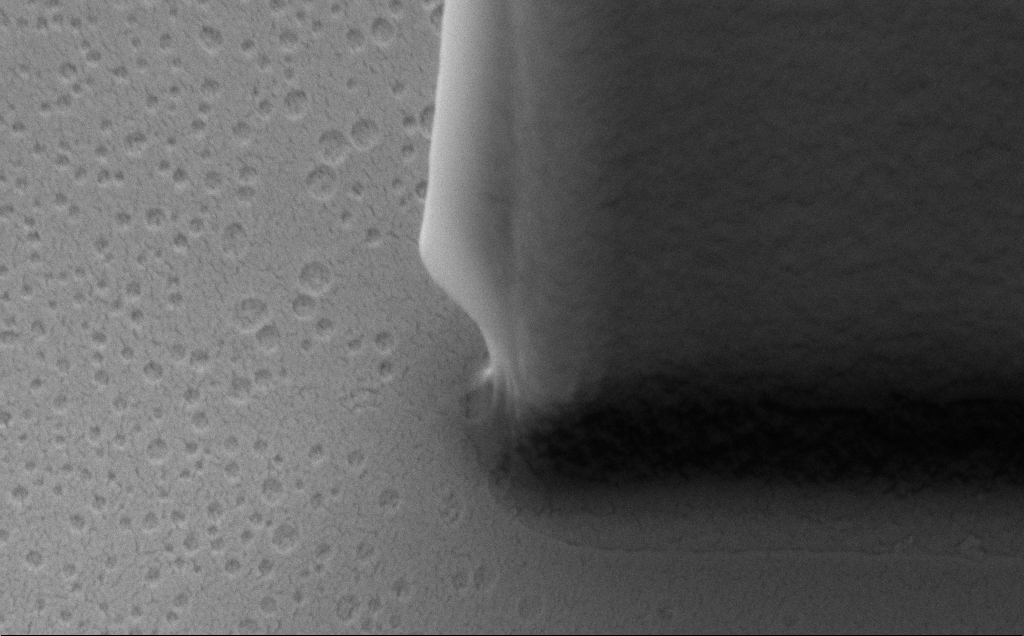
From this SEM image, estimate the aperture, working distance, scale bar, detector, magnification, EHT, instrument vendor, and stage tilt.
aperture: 30 µm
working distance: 9 mm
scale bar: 1000 nm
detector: SE2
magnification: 57.76 K X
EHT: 5 kV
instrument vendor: Zeiss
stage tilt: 40°